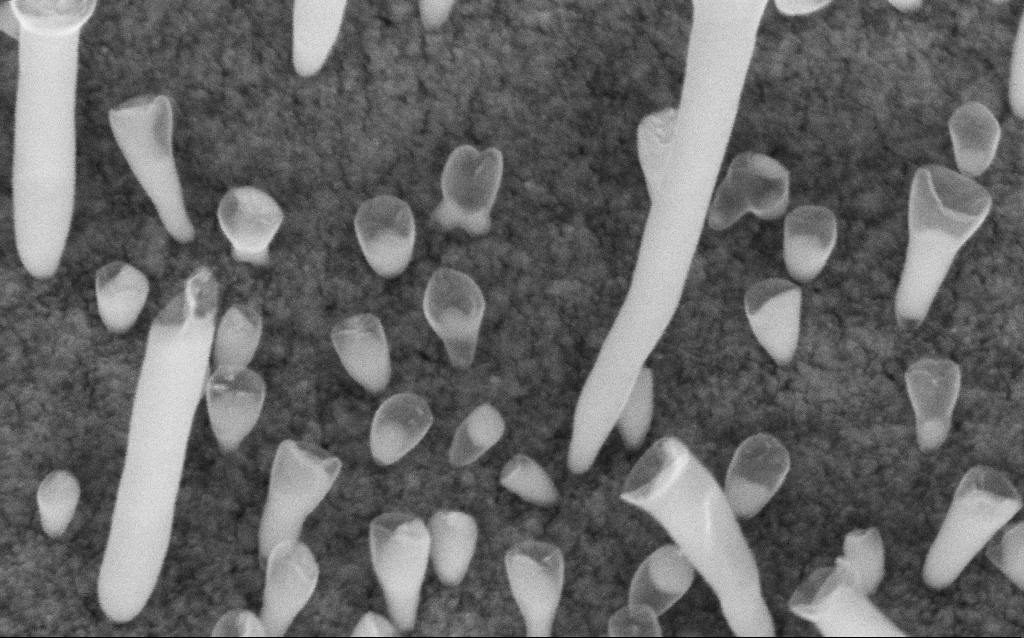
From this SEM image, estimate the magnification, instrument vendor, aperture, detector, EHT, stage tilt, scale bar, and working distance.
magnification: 200 K X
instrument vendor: Zeiss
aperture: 30 µm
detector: InLens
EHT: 5 kV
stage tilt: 45°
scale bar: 200 nm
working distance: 6.7 mm